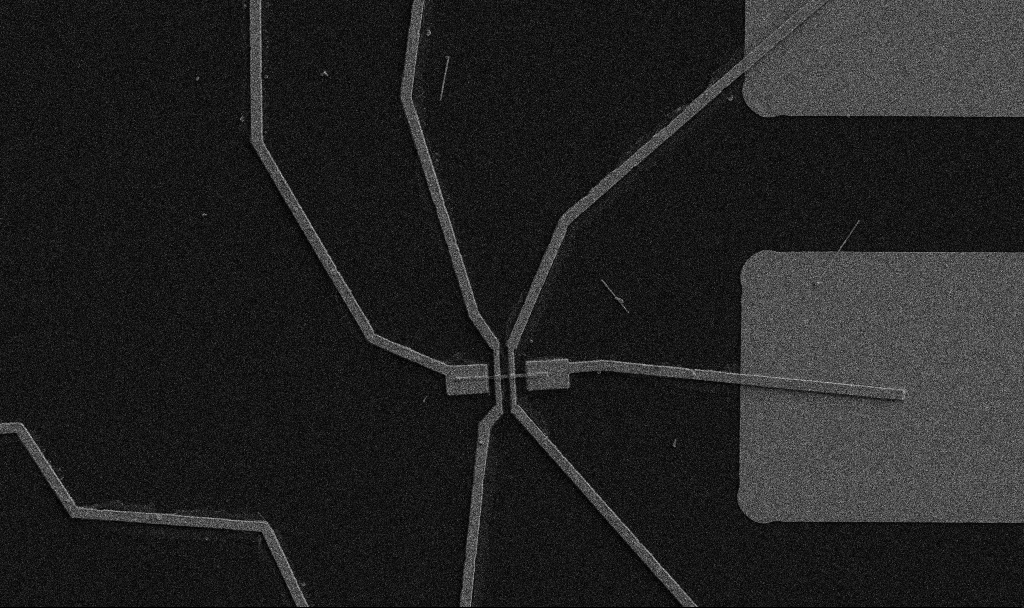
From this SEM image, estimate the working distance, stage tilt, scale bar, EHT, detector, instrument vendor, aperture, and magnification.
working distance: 10.7 mm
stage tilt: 0°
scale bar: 10000 nm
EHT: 5 kV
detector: SE2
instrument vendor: Zeiss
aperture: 30 µm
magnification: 5 K X